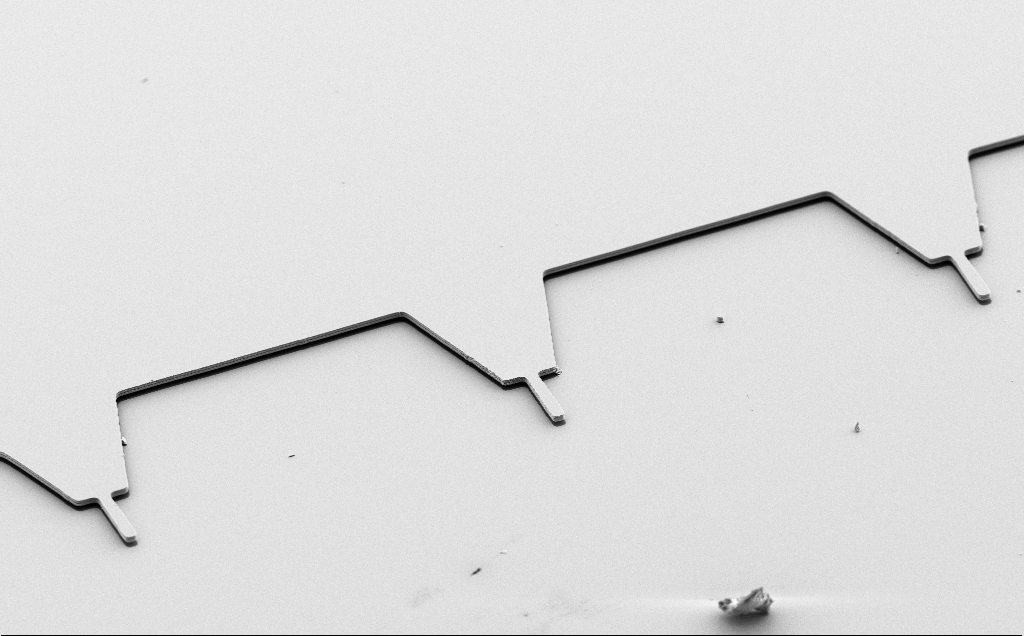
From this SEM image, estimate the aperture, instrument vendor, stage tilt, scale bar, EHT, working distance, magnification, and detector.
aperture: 30 µm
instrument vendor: Zeiss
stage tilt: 50°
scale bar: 20000 nm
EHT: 5 kV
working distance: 10 mm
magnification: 1.07 K X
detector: SE2